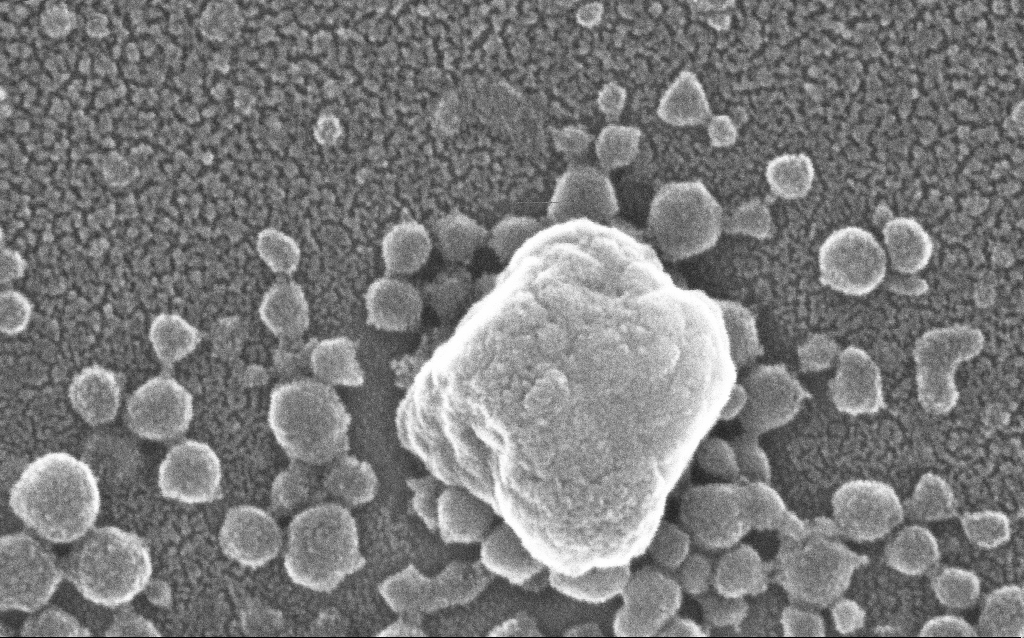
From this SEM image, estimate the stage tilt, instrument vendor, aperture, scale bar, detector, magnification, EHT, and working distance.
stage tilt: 0°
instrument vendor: Zeiss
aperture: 30 µm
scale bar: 100 nm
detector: InLens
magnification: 500 K X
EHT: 20 kV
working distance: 1.5 mm